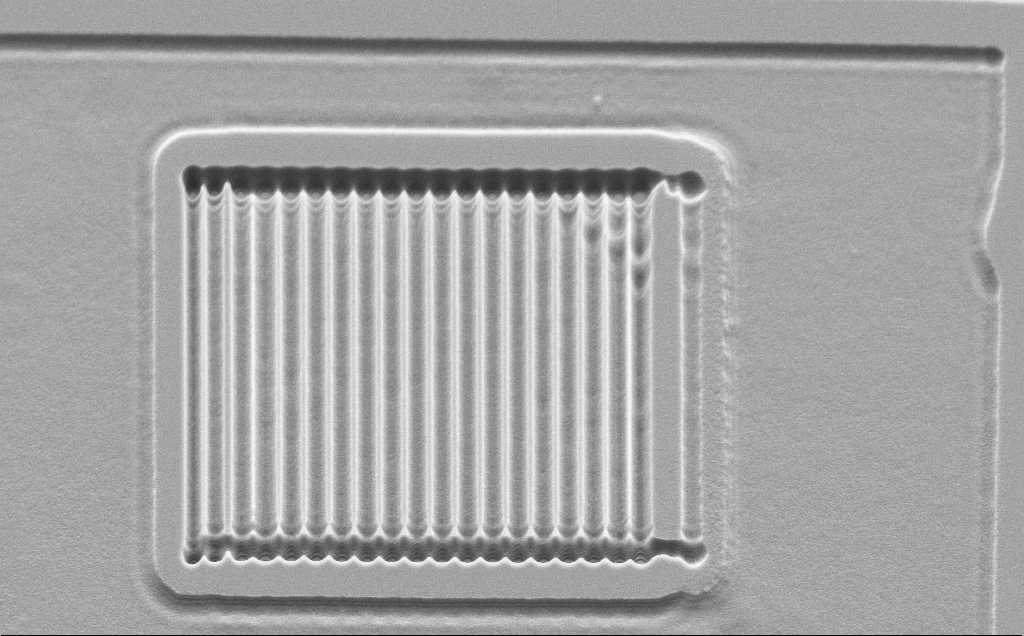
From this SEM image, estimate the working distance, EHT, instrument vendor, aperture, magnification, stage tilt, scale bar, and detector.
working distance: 10 mm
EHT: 5 kV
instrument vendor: Zeiss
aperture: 30 µm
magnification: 4.62 K X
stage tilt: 45°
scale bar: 10000 nm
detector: SE2